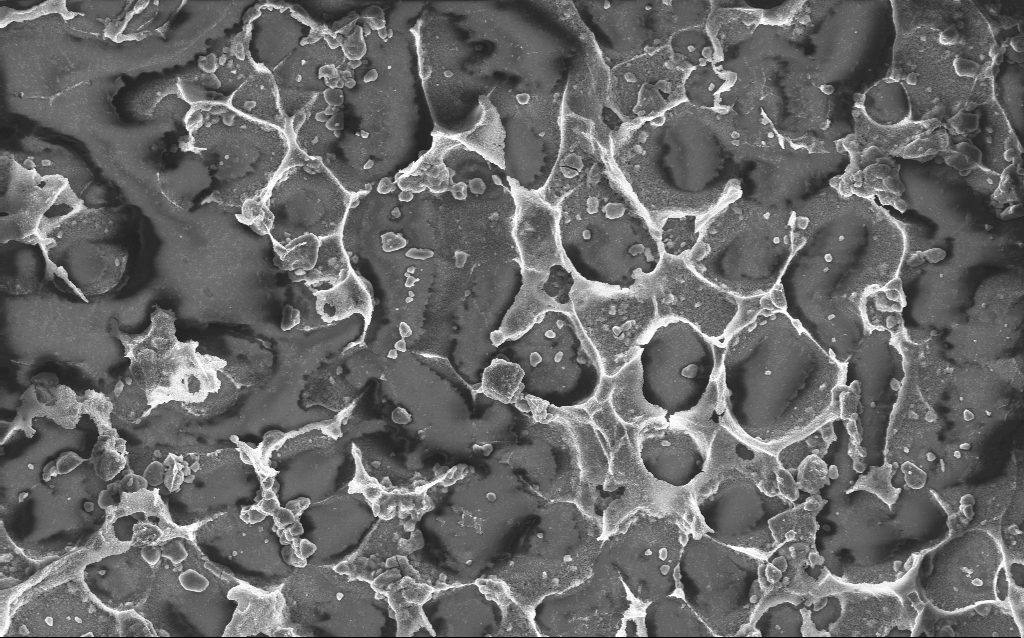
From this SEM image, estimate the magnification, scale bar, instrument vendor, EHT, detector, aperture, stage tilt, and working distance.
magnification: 1.69 K X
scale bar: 10000 nm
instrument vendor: Zeiss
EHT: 10 kV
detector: InLens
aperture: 30 µm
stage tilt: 0°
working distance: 2.8 mm